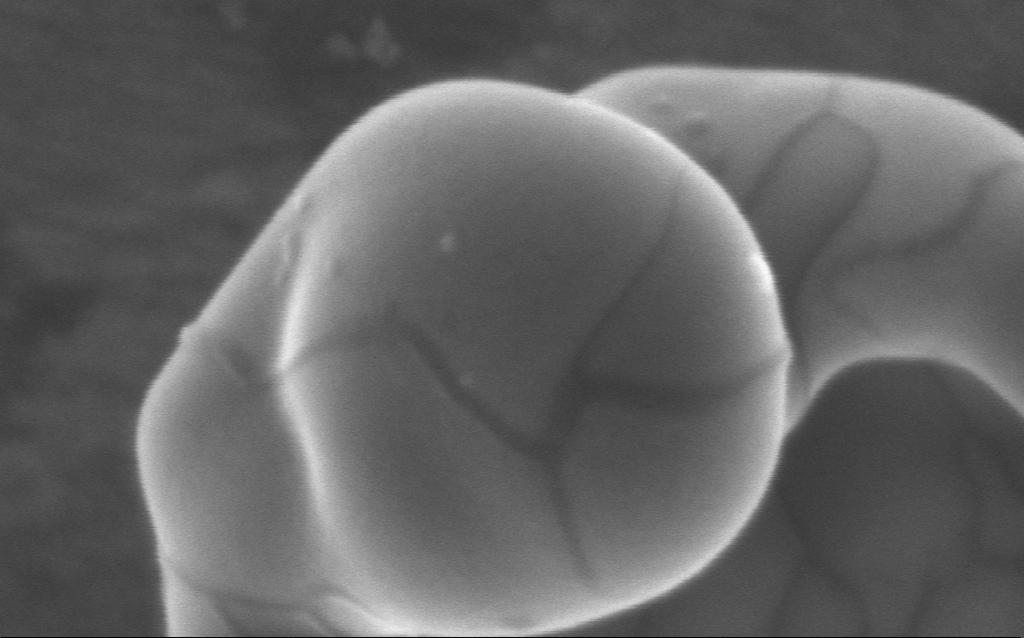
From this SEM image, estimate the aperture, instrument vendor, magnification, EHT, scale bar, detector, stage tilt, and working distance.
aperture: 30 µm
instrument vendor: Zeiss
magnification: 511.26 K X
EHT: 5 kV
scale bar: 100 nm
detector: InLens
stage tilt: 0°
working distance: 4 mm